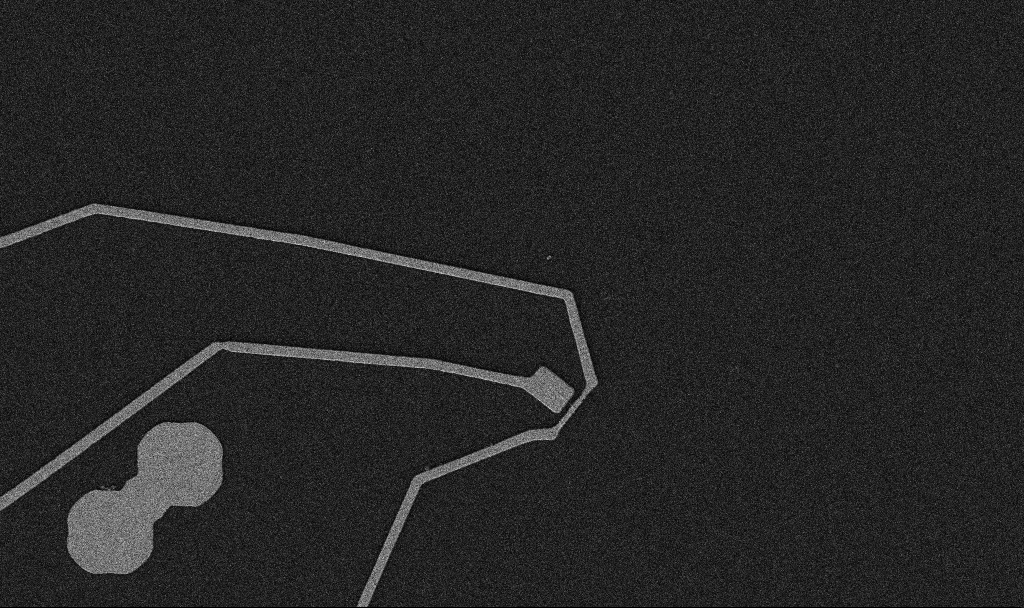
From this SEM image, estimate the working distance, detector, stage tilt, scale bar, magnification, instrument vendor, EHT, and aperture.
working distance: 10.7 mm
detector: SE2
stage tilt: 0°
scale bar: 10000 nm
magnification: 5 K X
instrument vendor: Zeiss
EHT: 5 kV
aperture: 30 µm